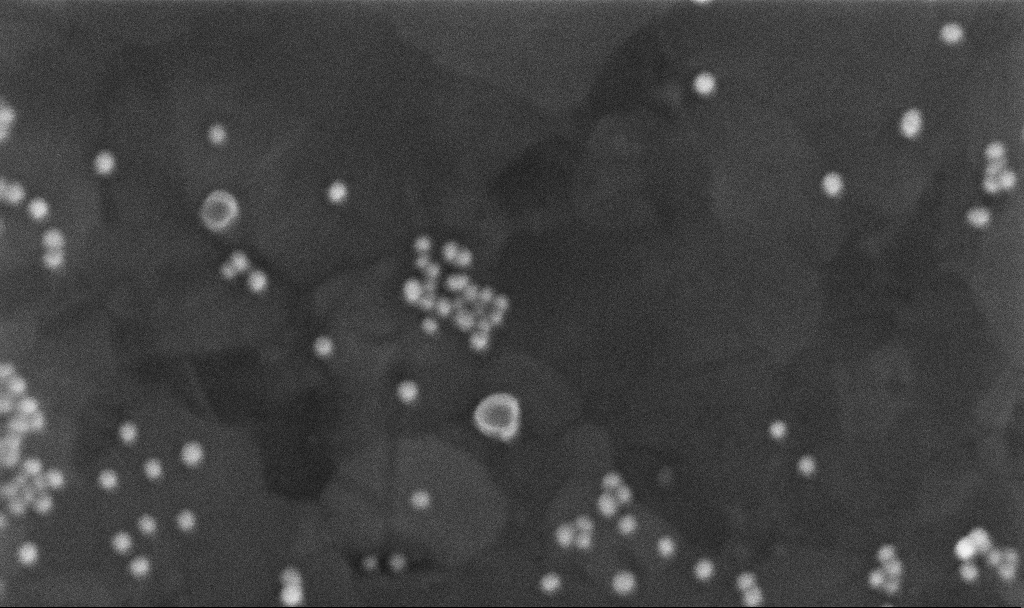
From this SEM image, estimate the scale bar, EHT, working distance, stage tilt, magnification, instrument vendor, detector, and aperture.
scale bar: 200 nm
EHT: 10 kV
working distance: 3.7 mm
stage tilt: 0°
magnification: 334.03 K X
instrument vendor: Zeiss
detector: InLens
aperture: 30 µm